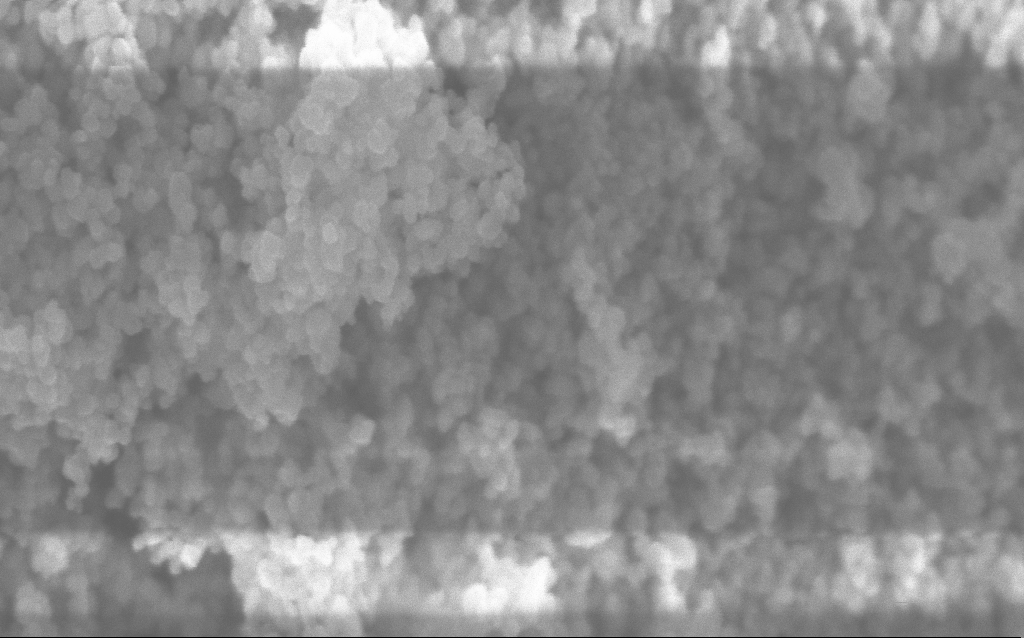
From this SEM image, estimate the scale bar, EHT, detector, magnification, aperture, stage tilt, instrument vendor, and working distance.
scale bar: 100 nm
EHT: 5 kV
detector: InLens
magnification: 211.33 K X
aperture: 30 µm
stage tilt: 0°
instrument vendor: Zeiss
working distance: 2.5 mm